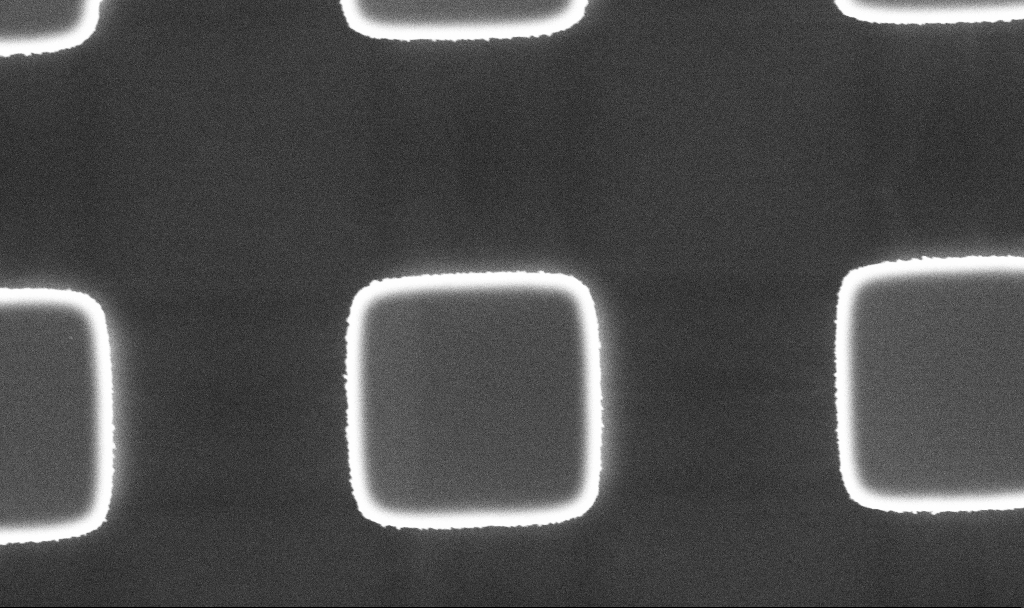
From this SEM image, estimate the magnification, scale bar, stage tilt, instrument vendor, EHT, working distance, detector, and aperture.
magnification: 22.54 K X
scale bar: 2000 nm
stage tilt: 0°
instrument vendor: Zeiss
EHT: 5 kV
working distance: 5.2 mm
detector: InLens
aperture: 30 µm